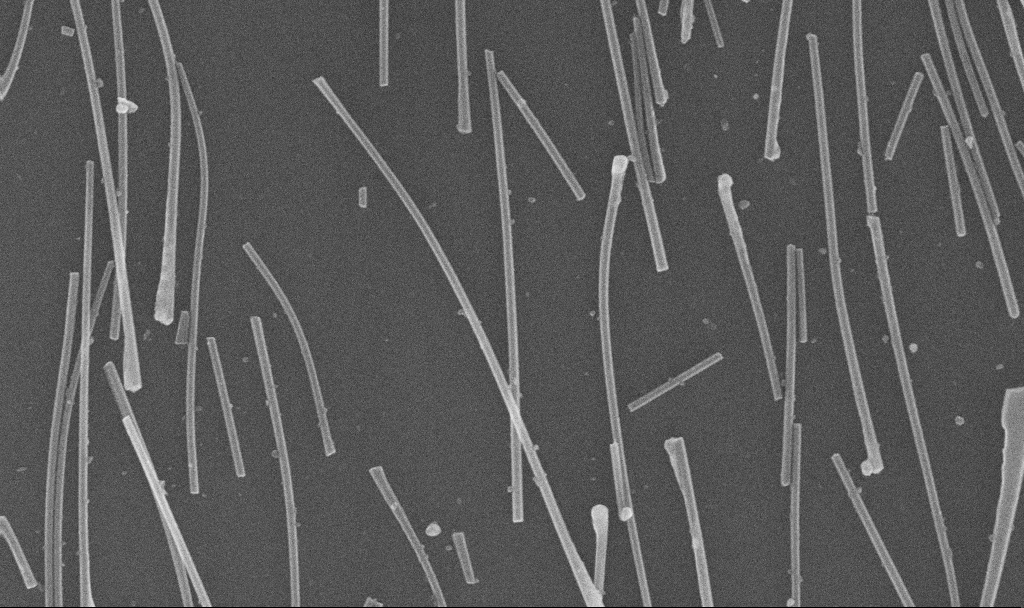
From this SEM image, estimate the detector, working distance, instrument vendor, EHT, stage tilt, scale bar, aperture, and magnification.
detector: InLens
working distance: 6.8 mm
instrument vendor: Zeiss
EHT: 20 kV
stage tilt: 0°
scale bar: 2000 nm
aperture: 30 µm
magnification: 30.88 K X